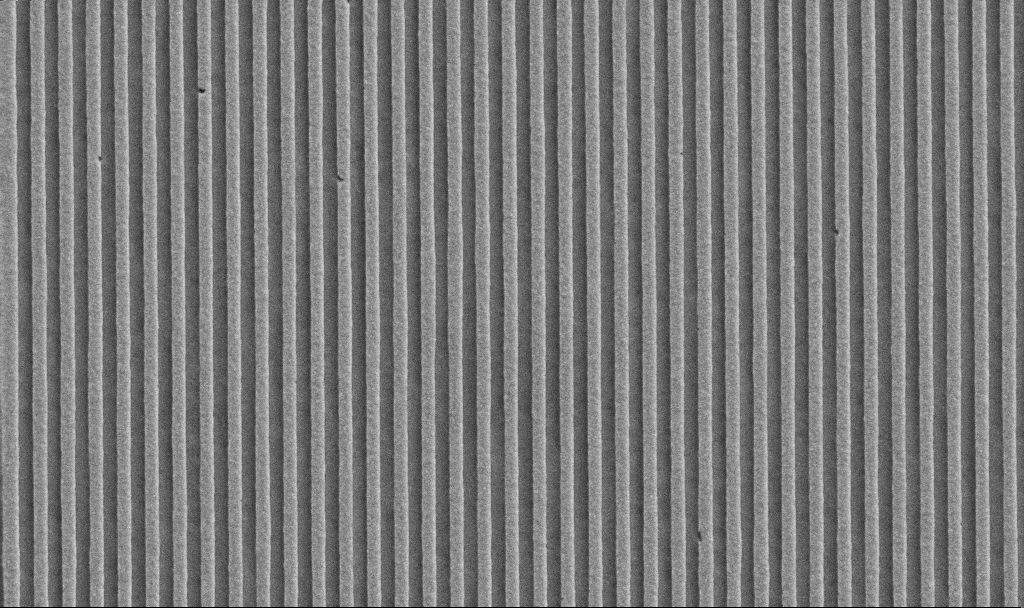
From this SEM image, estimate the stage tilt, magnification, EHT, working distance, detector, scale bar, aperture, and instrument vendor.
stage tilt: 0°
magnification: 25.09 K X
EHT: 10 kV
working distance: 5.6 mm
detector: SE2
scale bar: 1000 nm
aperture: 60 µm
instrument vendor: Zeiss